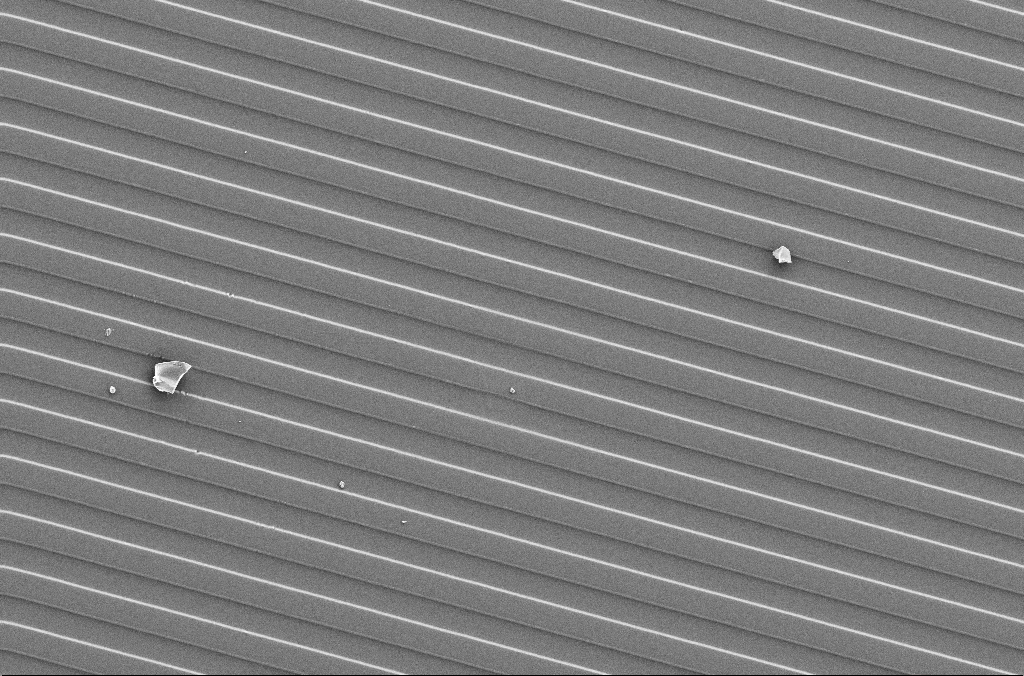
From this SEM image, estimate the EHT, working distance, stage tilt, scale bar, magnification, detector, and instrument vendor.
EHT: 5 kV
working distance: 4.1 mm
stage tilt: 0°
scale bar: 20000 nm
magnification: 1 K X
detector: SE2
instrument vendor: Zeiss